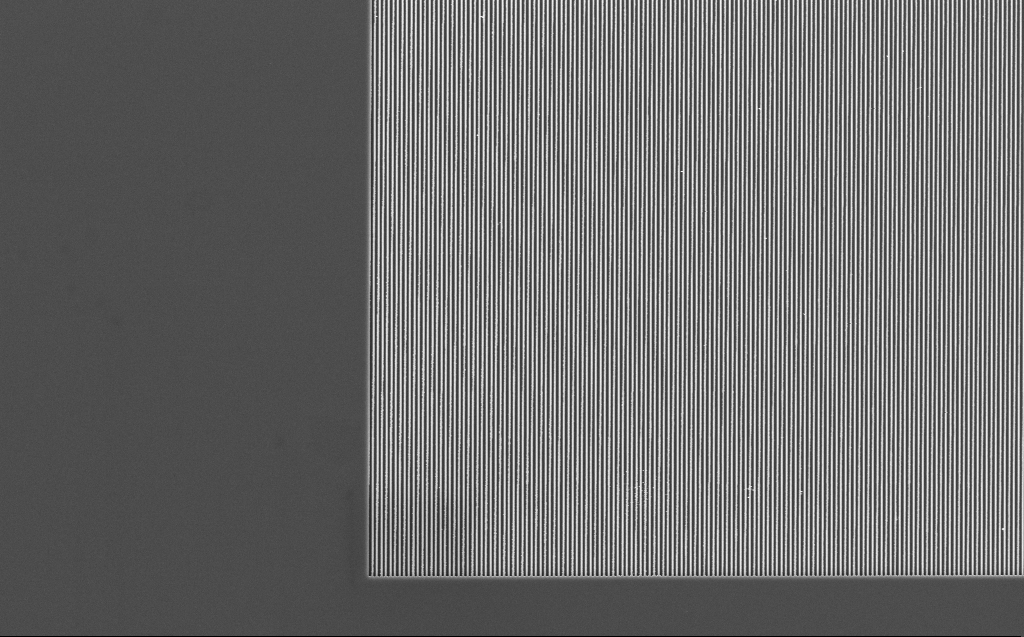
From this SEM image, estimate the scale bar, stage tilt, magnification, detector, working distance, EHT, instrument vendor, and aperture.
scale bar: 10000 nm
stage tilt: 0°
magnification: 6.7 K X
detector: InLens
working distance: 7 mm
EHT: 5 kV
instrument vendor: Zeiss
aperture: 30 µm